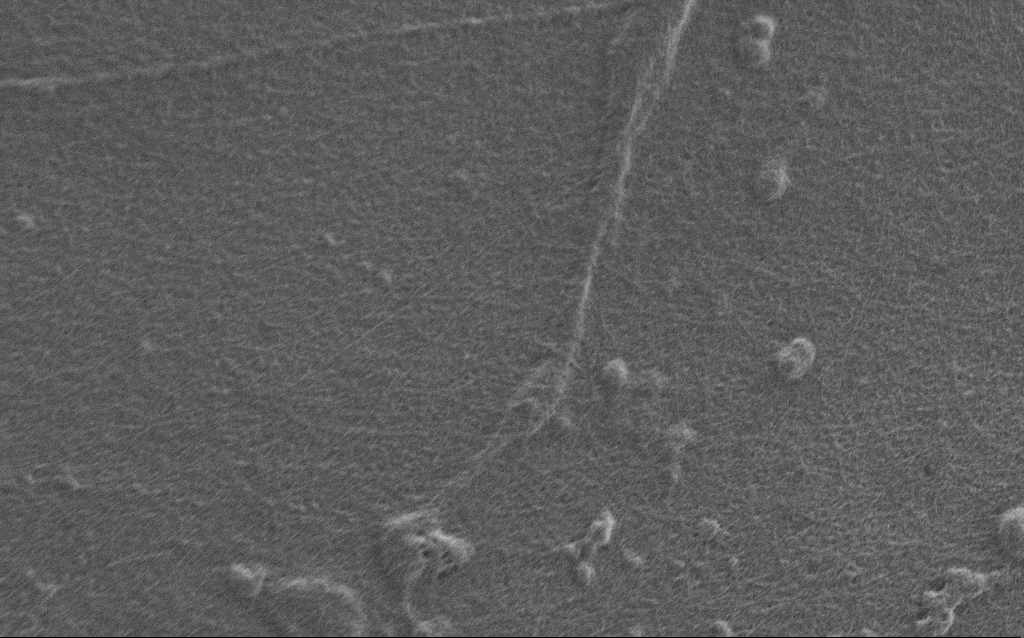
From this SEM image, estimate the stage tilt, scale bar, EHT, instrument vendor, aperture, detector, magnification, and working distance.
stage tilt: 0°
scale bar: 2000 nm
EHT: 0.9 kV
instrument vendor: Zeiss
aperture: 30 µm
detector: SE2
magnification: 10 K X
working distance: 6 mm